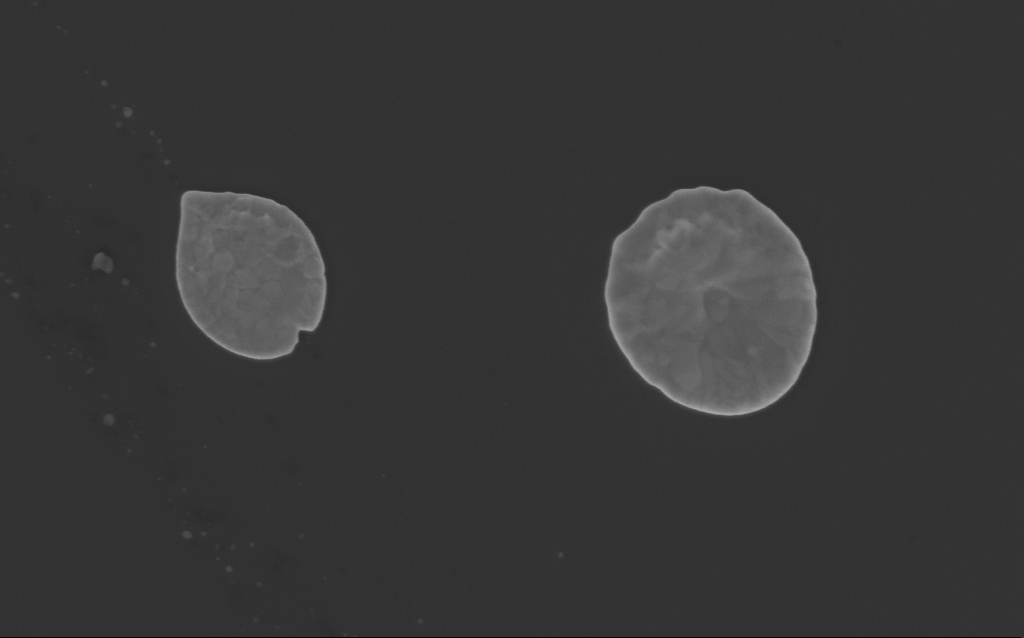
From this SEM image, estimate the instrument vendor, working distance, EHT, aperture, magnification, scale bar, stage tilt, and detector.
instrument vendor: Zeiss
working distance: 4 mm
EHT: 3 kV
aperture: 30 µm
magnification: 48.79 K X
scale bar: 1000 nm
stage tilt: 0°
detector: InLens